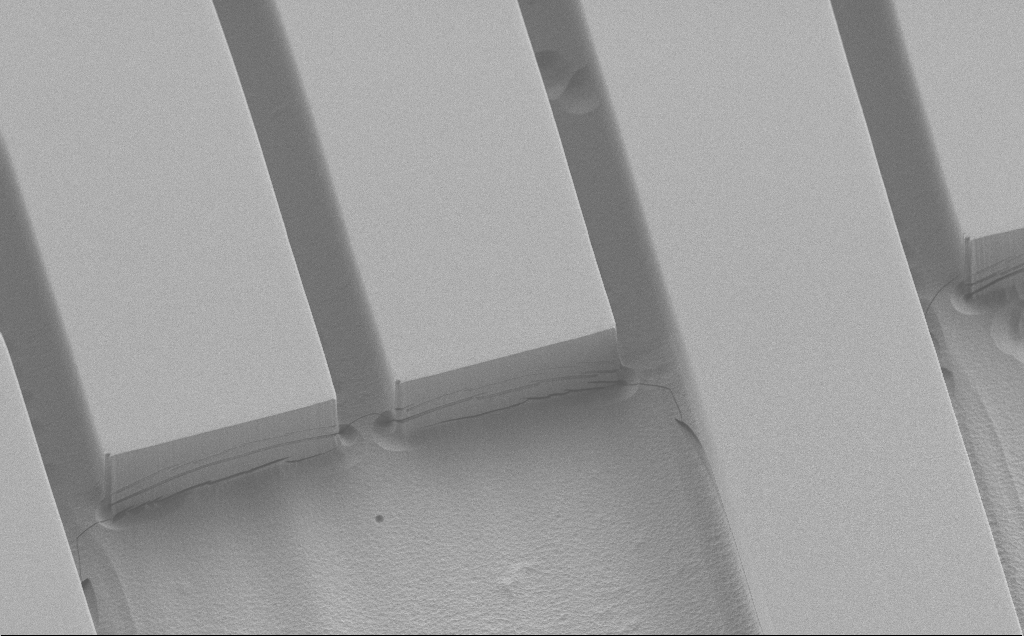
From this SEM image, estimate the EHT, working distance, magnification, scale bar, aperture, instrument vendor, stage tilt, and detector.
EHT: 1.2 kV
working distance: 6 mm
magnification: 1.02 K X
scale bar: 20000 nm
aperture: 30 µm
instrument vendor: Zeiss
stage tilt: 30°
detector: SE2